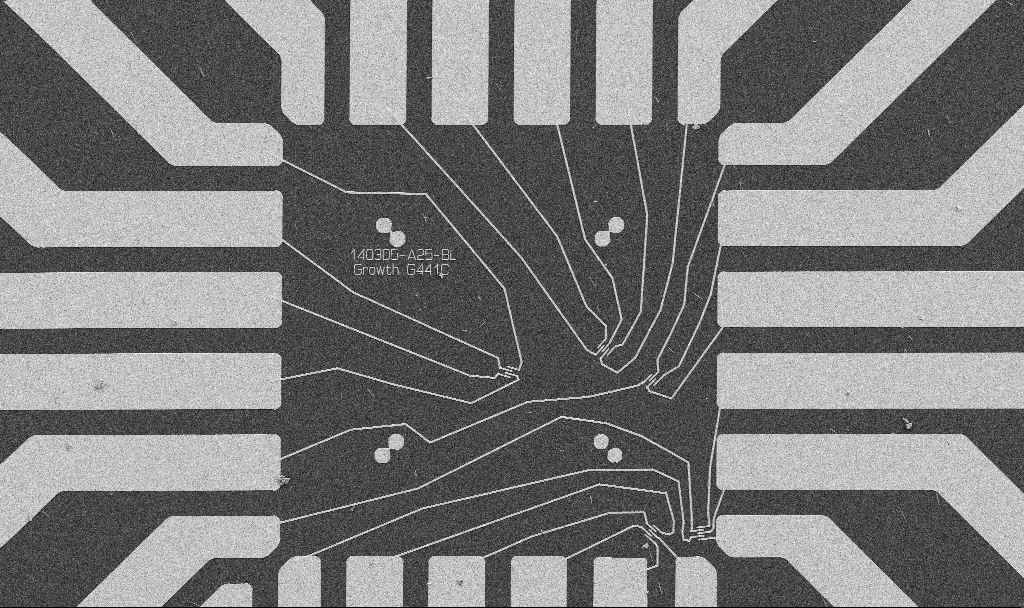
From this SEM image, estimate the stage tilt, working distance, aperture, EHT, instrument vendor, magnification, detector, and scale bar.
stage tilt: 0°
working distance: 10.7 mm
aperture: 30 µm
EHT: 5 kV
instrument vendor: Zeiss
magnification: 1 K X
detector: SE2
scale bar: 20000 nm